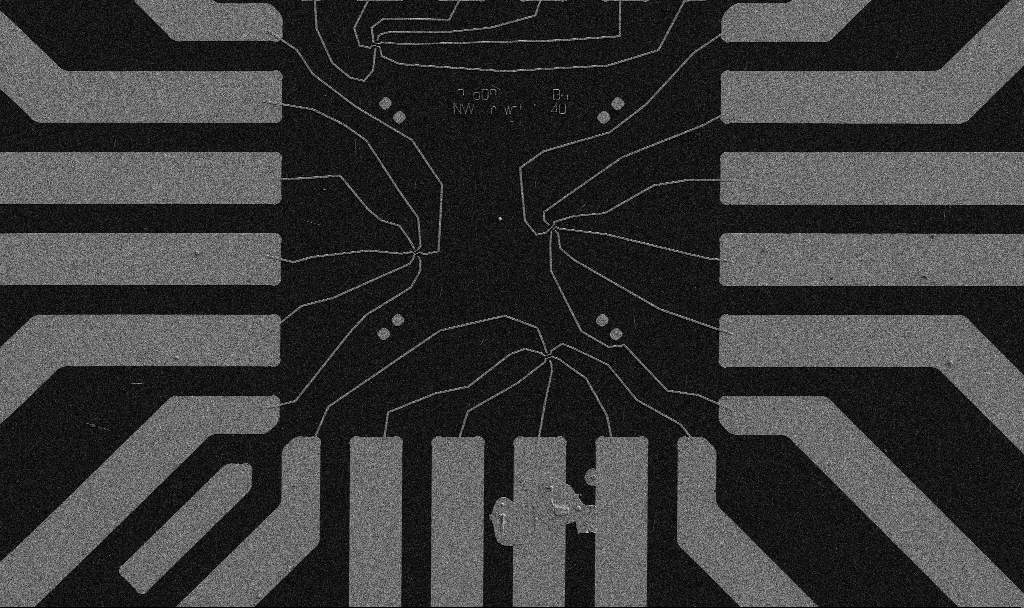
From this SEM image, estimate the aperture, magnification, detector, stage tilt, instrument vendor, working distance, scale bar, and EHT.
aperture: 30 µm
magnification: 1 K X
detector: SE2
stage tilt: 0°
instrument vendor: Zeiss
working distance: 10.7 mm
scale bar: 20000 nm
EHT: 5 kV